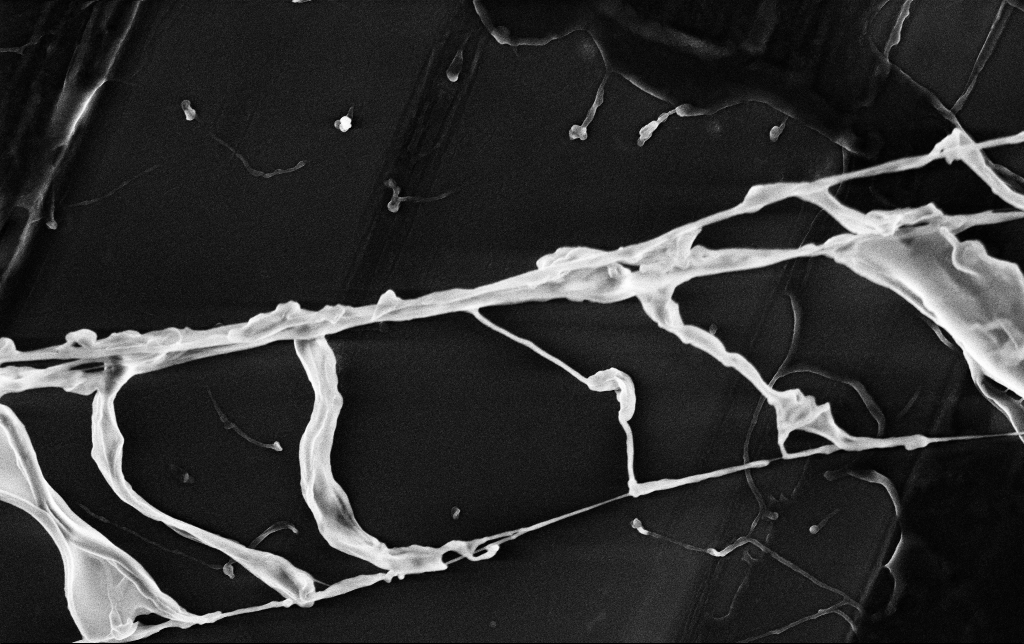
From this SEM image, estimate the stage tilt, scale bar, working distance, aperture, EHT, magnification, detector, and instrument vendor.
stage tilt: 0°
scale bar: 1000 nm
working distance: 3.5 mm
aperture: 30 µm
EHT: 3 kV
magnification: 23.28 K X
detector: InLens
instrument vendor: Zeiss